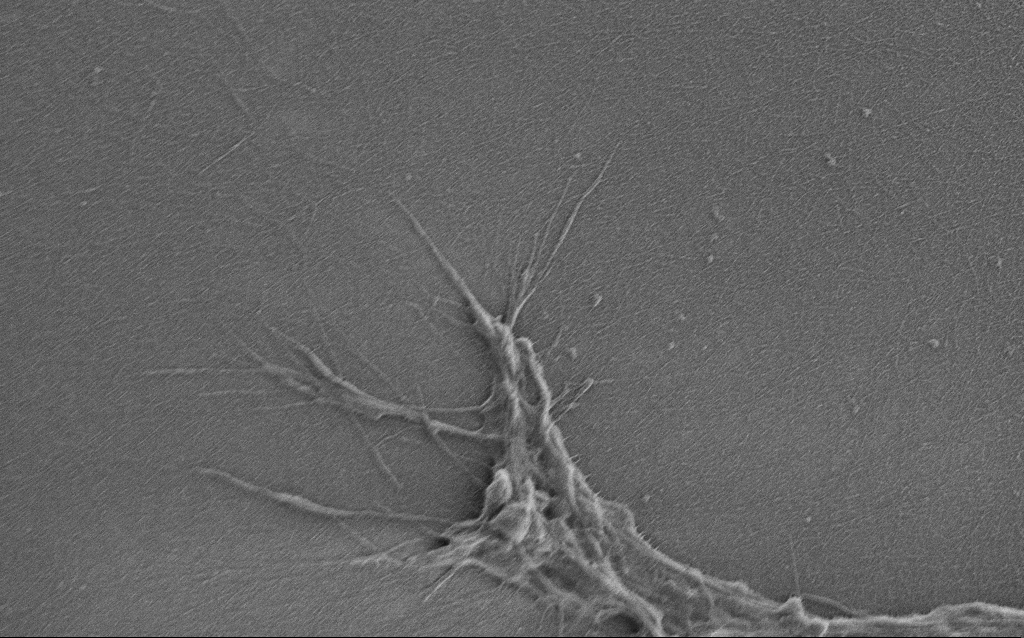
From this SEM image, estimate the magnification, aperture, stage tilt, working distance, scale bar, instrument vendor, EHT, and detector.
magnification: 7.5 K X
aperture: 30 µm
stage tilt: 0°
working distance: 6 mm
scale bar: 2000 nm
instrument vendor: Zeiss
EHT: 0.9 kV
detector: SE2